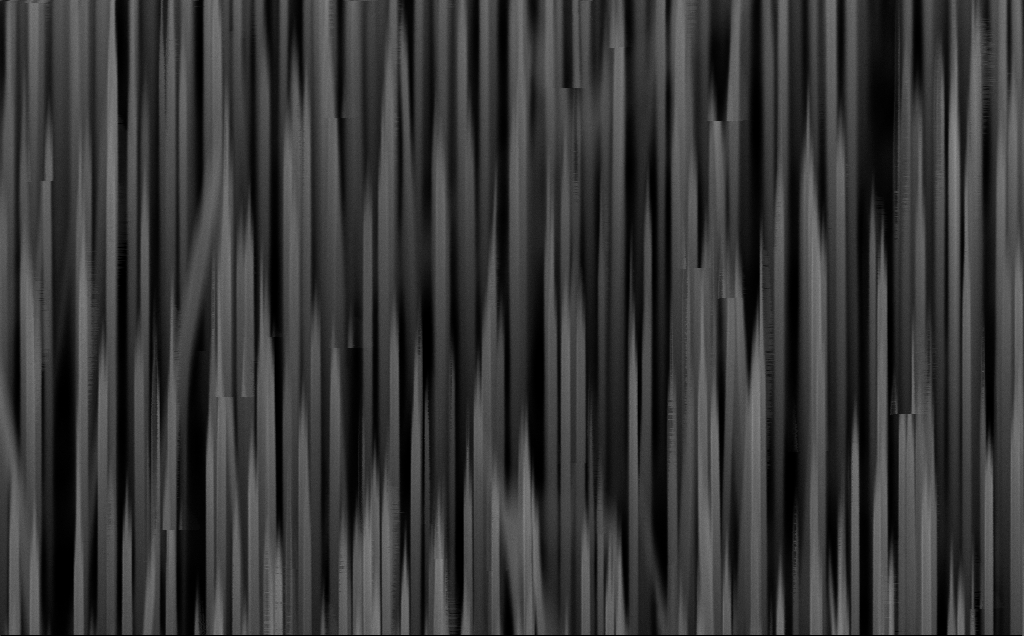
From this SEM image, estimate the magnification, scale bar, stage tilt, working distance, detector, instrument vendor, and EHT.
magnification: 54.05 K X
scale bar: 1000 nm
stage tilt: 45°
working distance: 9 mm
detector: InLens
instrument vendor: Zeiss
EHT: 10 kV